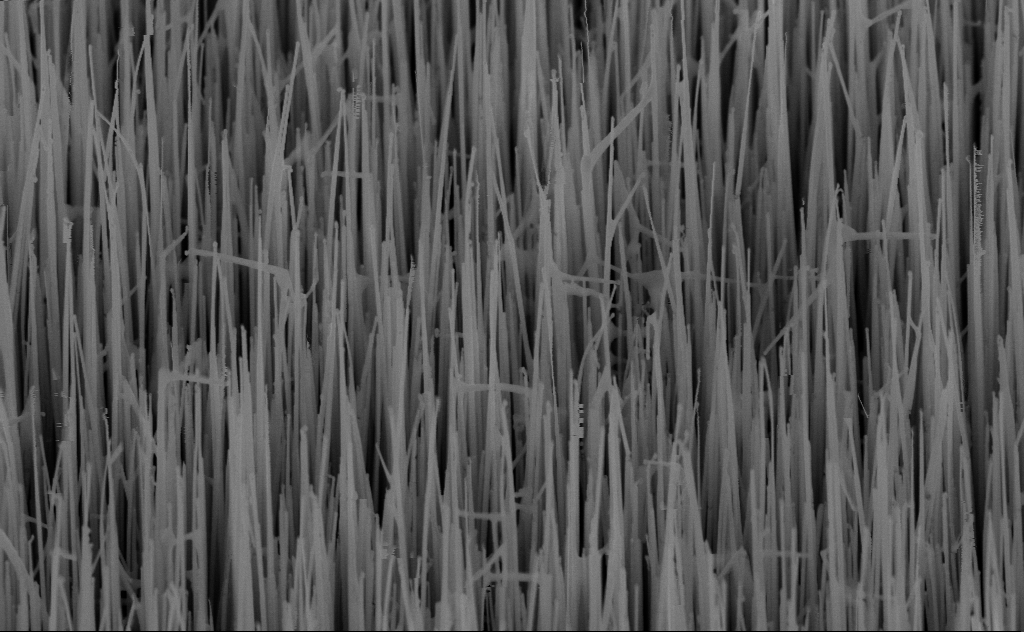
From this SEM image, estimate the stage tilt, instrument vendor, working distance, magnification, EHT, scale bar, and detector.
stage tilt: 45°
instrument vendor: Zeiss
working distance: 6 mm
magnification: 40 K X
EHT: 10 kV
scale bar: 1000 nm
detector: InLens